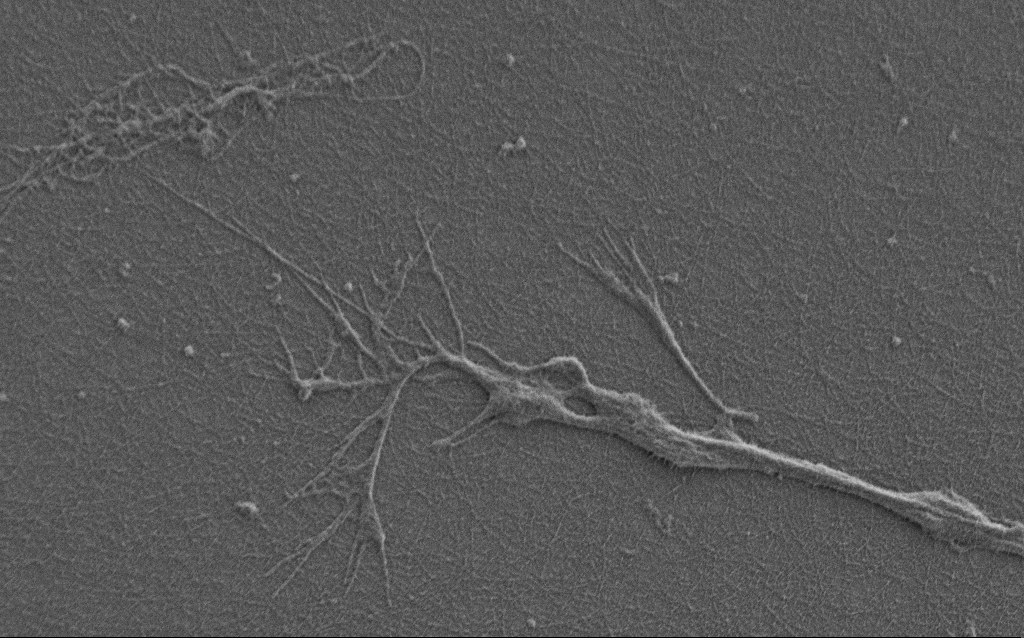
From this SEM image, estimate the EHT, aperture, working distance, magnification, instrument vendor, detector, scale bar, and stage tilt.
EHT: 0.9 kV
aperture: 30 µm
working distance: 6 mm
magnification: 10 K X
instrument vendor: Zeiss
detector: SE2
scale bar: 2000 nm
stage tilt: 0°